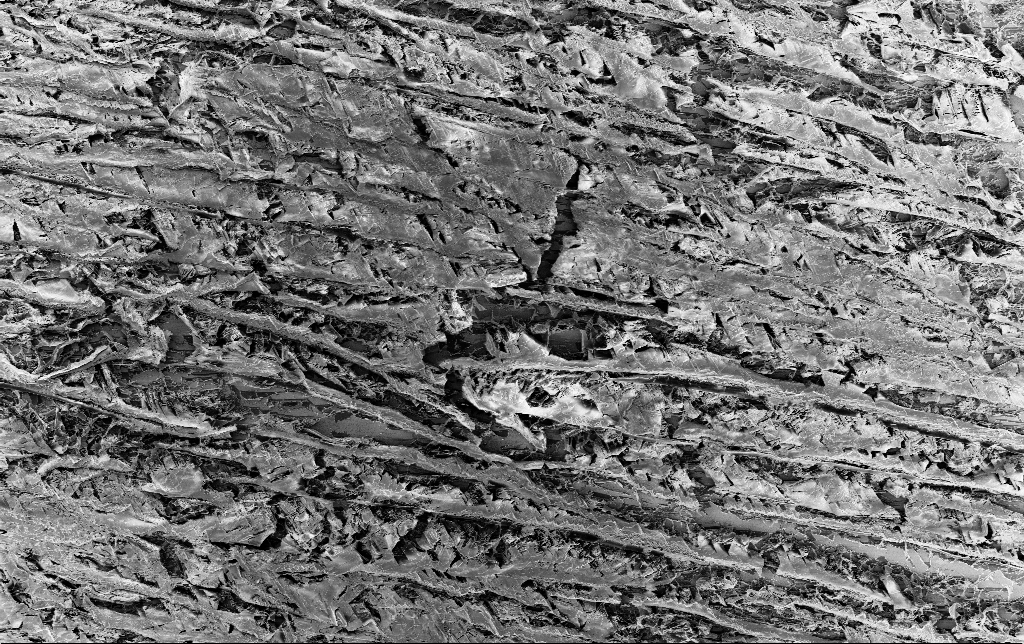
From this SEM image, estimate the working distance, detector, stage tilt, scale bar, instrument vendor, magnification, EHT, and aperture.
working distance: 3.2 mm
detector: InLens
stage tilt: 0°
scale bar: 20000 nm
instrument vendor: Zeiss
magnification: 0.821 K X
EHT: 1.5 kV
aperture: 30 µm